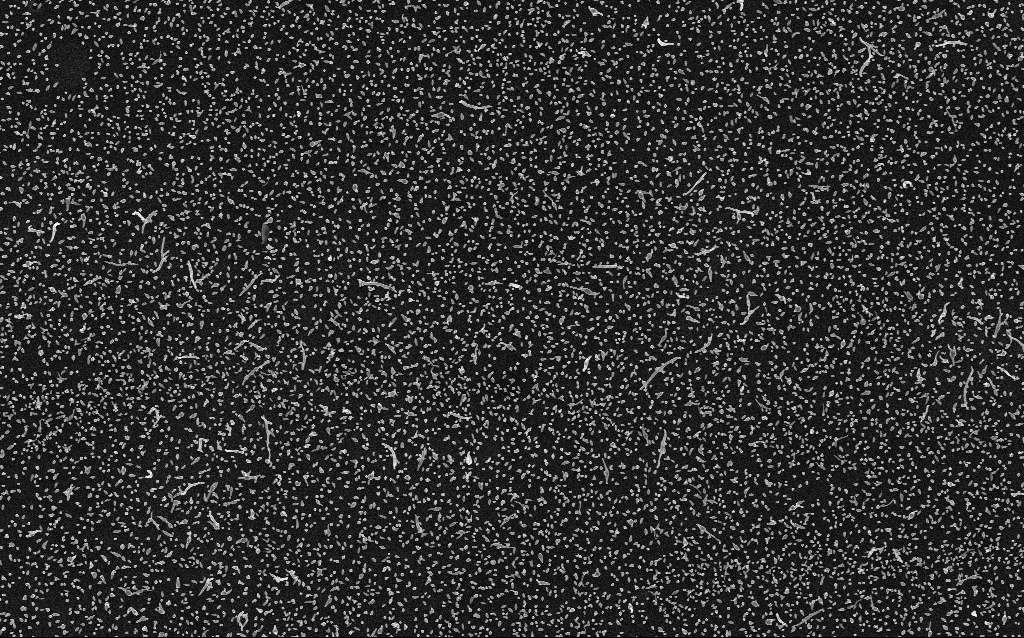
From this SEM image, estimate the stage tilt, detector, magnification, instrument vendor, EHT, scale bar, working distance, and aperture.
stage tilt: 0°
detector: InLens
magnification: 10 K X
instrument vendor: Zeiss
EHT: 5 kV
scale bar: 2000 nm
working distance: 2.9 mm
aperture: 30 µm